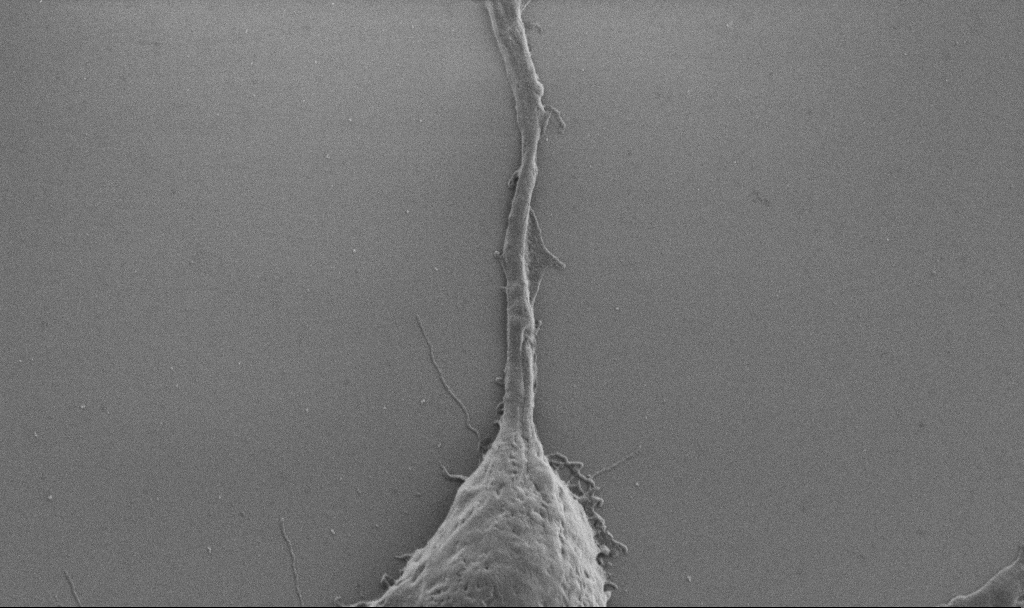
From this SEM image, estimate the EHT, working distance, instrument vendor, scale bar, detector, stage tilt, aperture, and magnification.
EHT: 1 kV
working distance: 6.9 mm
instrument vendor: Zeiss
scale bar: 2000 nm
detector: SE2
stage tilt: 0°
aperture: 30 µm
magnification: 10 K X